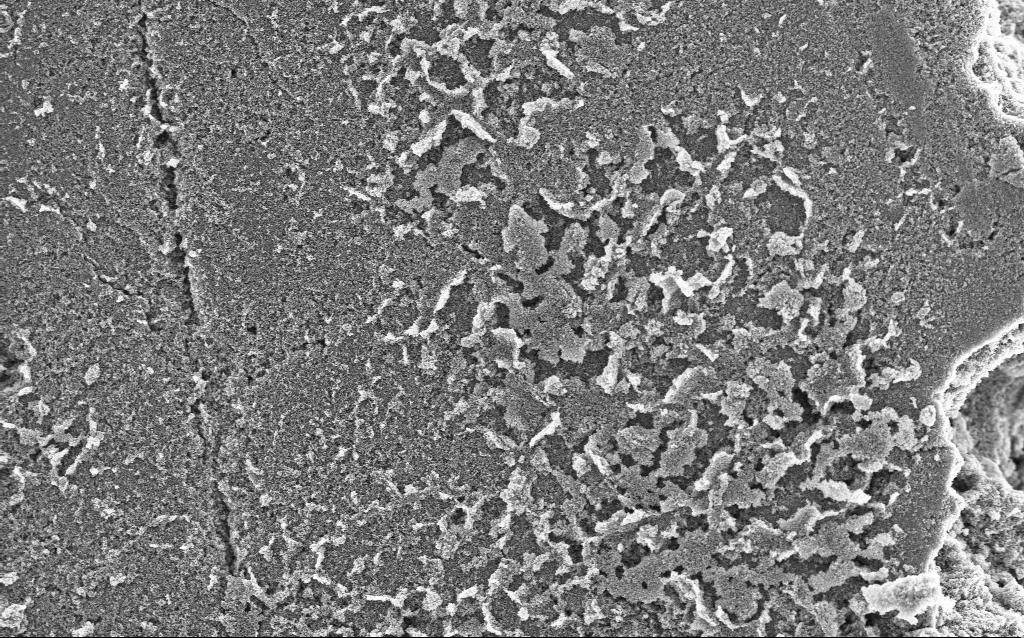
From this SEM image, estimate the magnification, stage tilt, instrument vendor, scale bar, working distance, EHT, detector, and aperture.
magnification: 6.42 K X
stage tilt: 0°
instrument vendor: Zeiss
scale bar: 10000 nm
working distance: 4.4 mm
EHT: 5 kV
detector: InLens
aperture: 30 µm